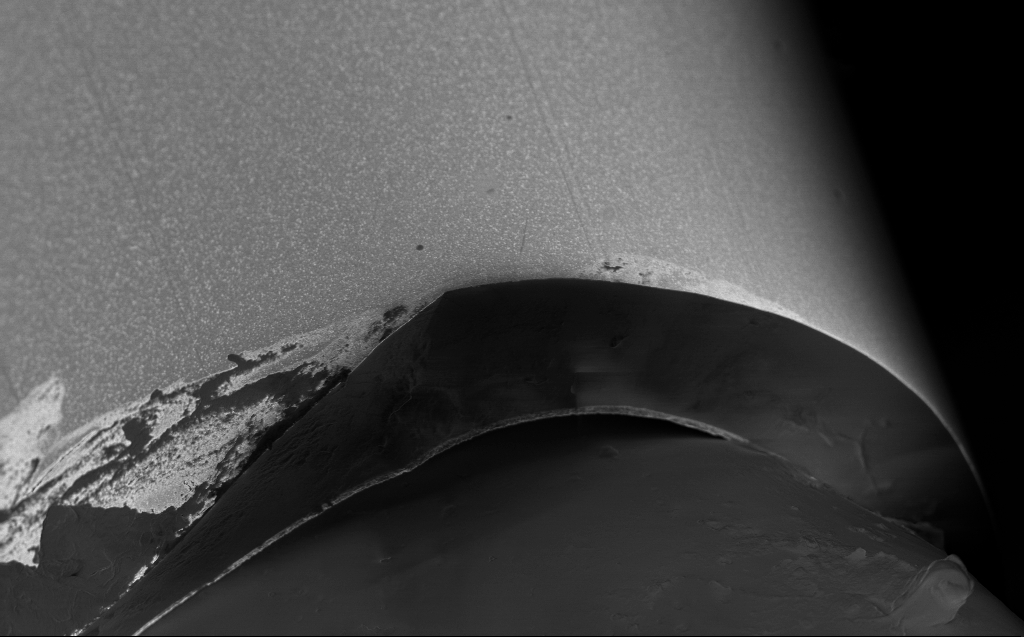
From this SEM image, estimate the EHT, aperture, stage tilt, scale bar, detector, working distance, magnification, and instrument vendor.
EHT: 0.8 kV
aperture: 30 µm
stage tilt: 45°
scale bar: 10000 nm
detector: InLens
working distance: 3 mm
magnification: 6.44 K X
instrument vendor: Zeiss